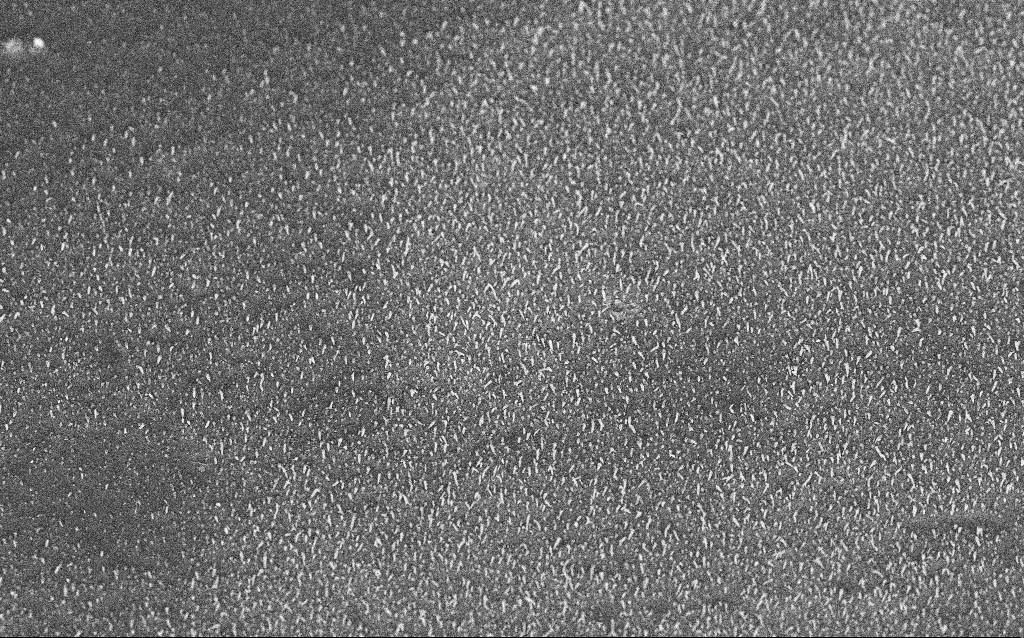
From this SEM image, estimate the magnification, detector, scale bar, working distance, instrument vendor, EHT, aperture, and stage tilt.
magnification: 10 K X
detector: InLens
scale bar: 2000 nm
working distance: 6.5 mm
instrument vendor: Zeiss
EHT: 5 kV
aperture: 30 µm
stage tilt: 45°